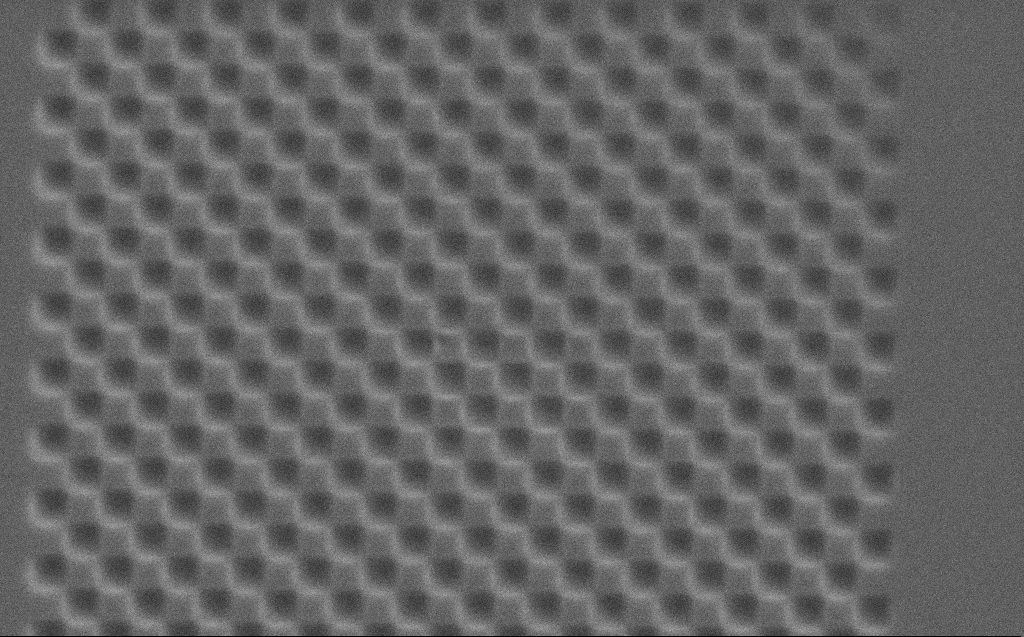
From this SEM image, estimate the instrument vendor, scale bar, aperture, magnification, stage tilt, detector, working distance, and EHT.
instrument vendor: Zeiss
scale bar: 2000 nm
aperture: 30 µm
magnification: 12.2 K X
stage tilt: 0°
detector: SE2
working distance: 5 mm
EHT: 5 kV